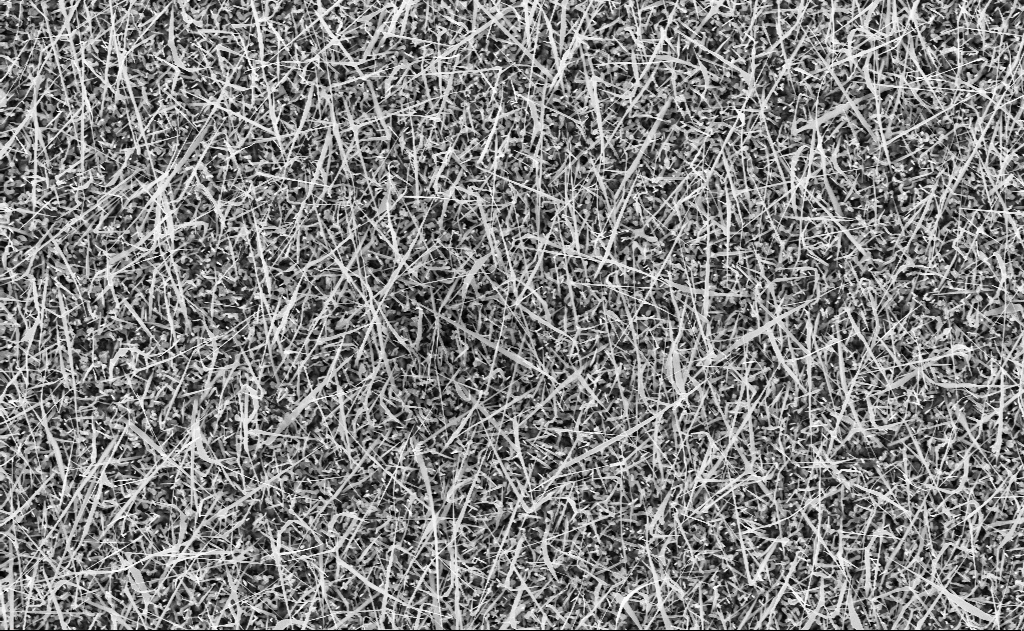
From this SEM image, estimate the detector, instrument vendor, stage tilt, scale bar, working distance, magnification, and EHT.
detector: InLens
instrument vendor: Zeiss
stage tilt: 0°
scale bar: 2000 nm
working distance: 10 mm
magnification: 10 K X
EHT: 10 kV